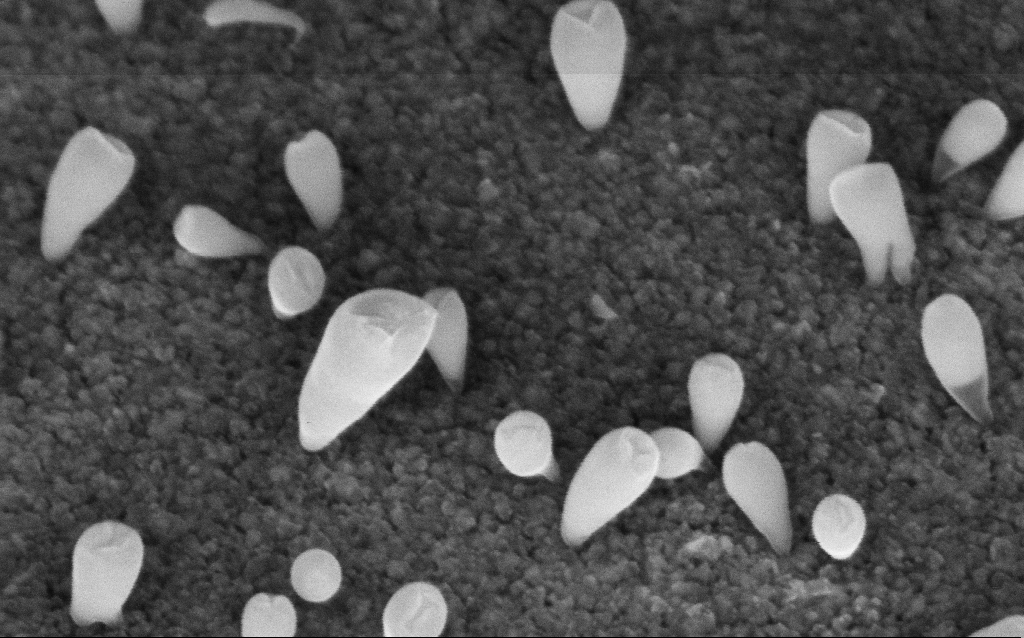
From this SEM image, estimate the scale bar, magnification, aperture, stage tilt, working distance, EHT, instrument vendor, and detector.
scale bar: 200 nm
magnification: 236.74 K X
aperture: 30 µm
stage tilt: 42.7°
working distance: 6 mm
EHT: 5 kV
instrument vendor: Zeiss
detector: InLens